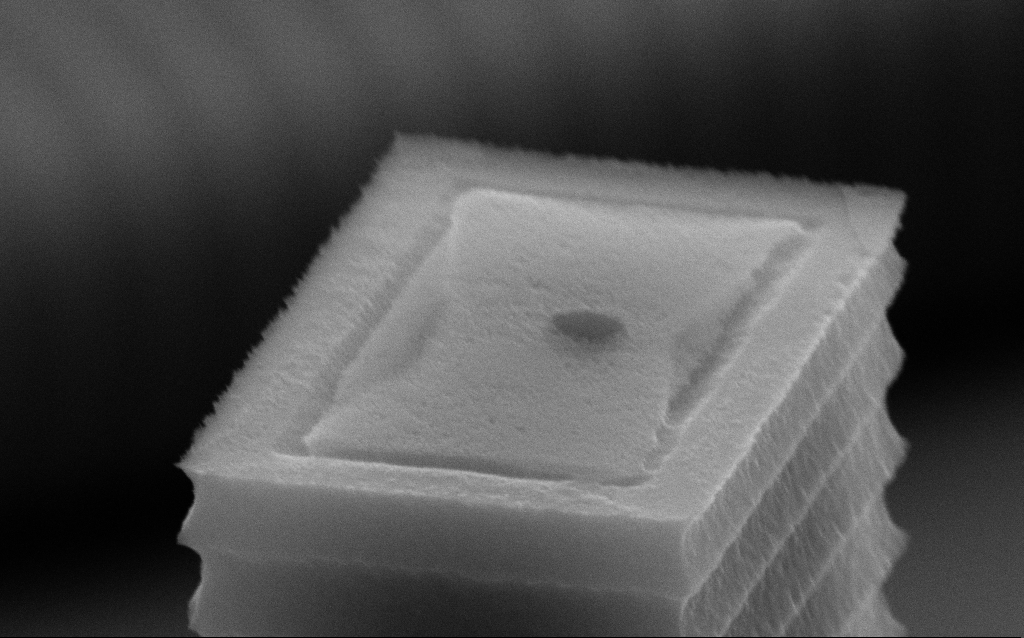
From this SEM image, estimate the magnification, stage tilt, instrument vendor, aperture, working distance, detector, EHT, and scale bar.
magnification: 107.79 K X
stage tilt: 70°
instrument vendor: Zeiss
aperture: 30 µm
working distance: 7.3 mm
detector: SE2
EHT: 10 kV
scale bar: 200 nm